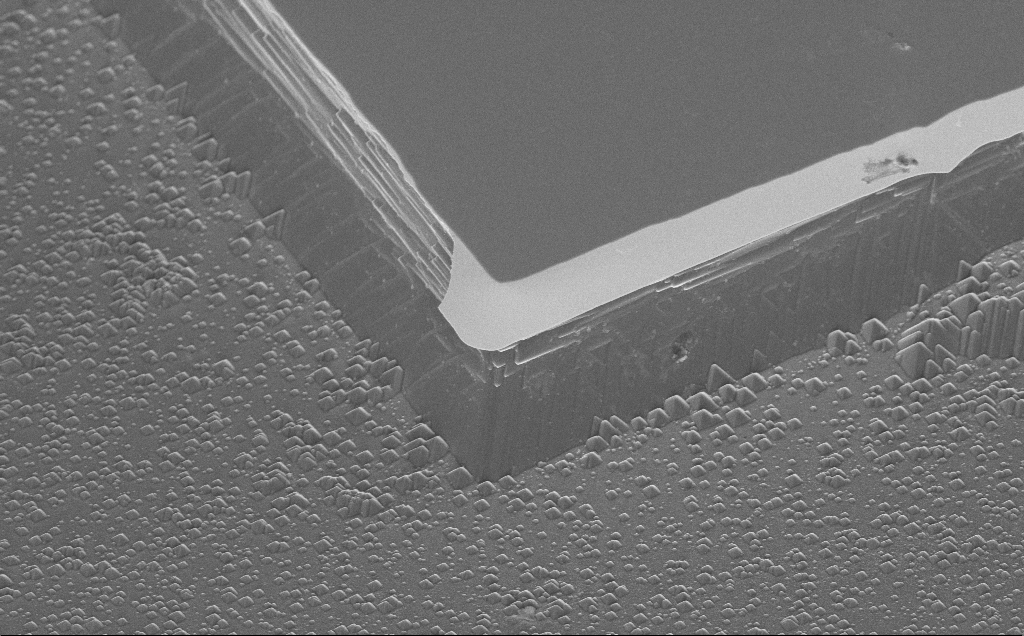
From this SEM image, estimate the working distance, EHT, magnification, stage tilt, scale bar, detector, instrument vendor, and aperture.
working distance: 13 mm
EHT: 5 kV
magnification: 4.3 K X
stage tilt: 45°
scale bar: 10000 nm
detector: InLens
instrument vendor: Zeiss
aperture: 30 µm